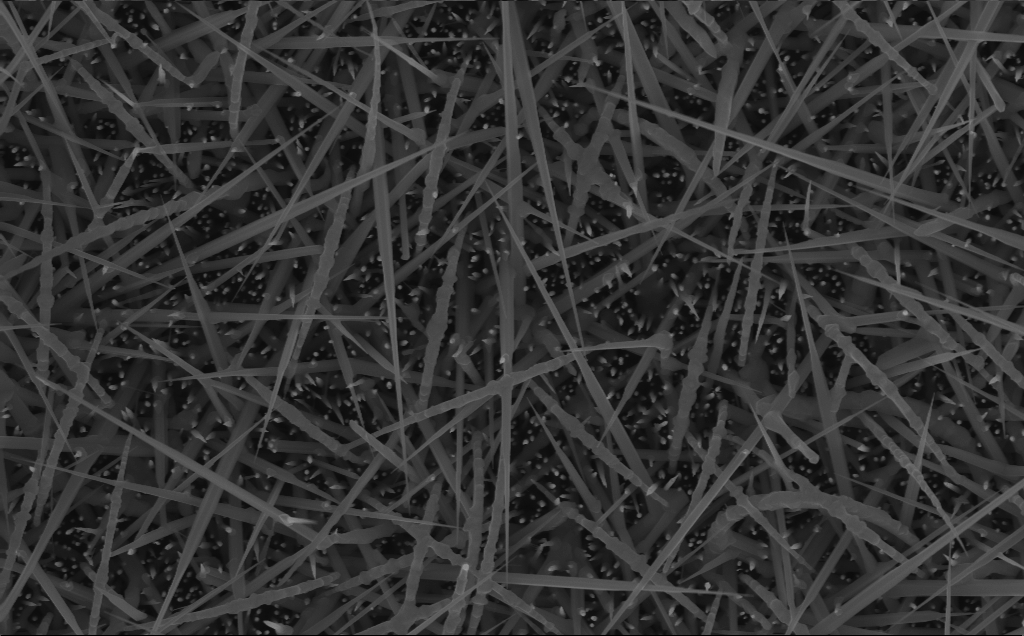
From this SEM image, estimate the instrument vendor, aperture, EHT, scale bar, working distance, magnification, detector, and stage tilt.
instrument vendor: Zeiss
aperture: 30 µm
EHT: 10 kV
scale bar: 1000 nm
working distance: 6 mm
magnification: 40 K X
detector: InLens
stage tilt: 0°